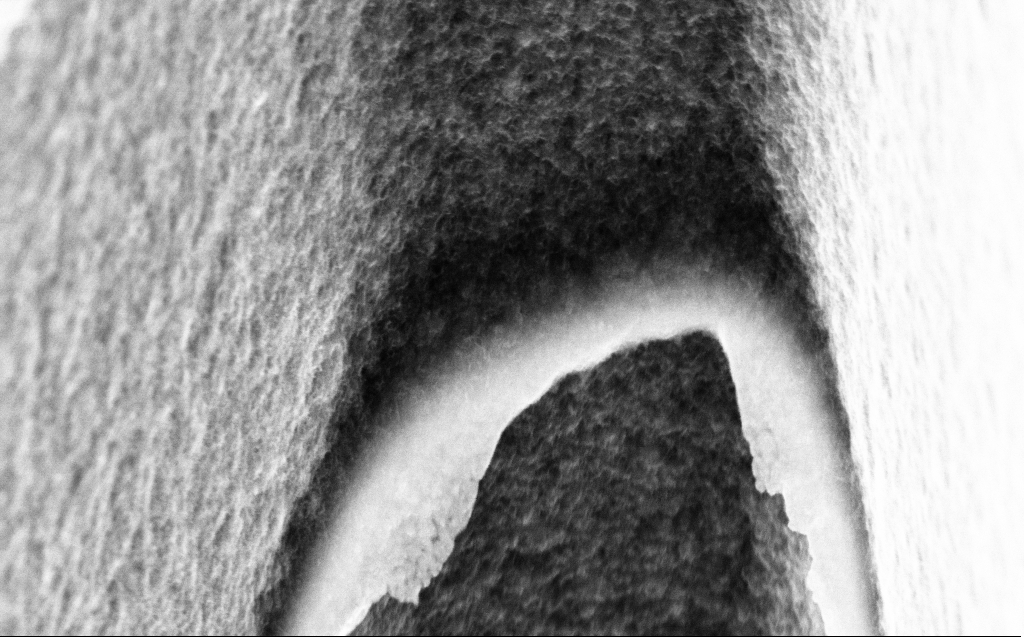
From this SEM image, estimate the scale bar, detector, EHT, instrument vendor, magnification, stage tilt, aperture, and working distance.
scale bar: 200 nm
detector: InLens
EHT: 10 kV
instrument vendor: Zeiss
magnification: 77.22 K X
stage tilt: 45°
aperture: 30 µm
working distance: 4 mm